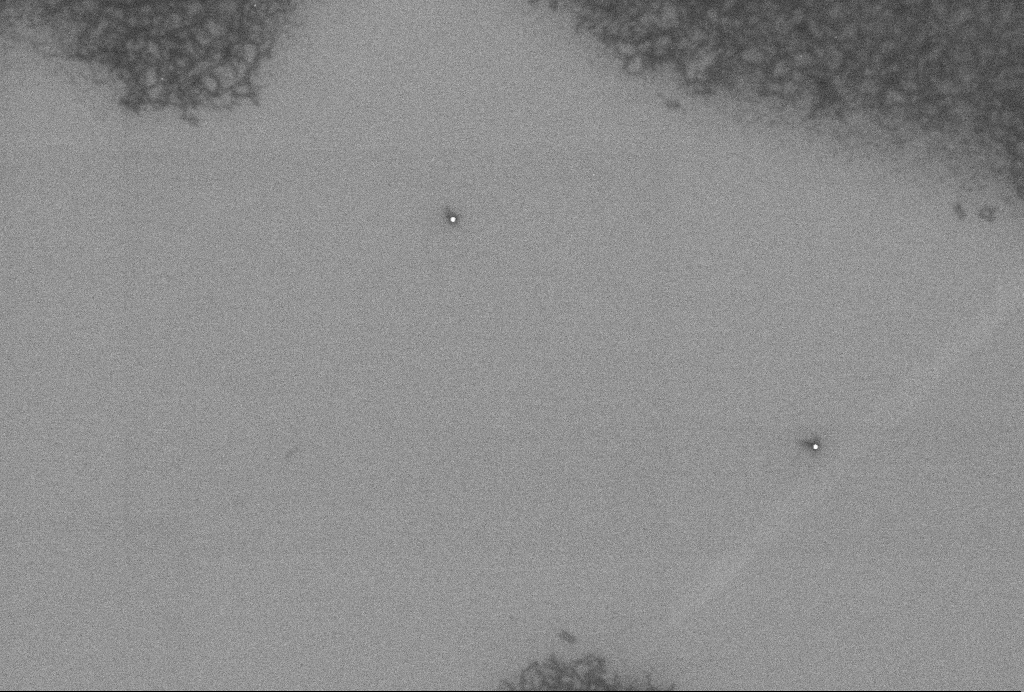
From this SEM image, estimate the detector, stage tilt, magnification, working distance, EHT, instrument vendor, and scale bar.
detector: InLens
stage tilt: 0°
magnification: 57.52 K X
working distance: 3.3 mm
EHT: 2 kV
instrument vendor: Zeiss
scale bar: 1000 nm